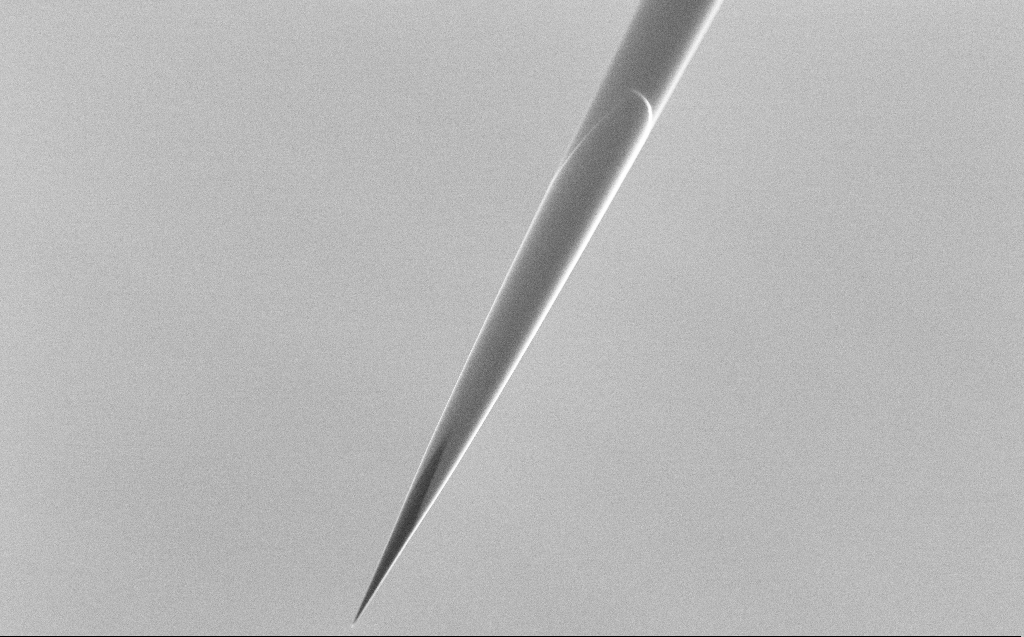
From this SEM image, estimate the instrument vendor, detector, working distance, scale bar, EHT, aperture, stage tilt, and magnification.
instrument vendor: Zeiss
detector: SE2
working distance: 6 mm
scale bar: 20000 nm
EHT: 5 kV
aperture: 30 µm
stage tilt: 45°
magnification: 1 K X